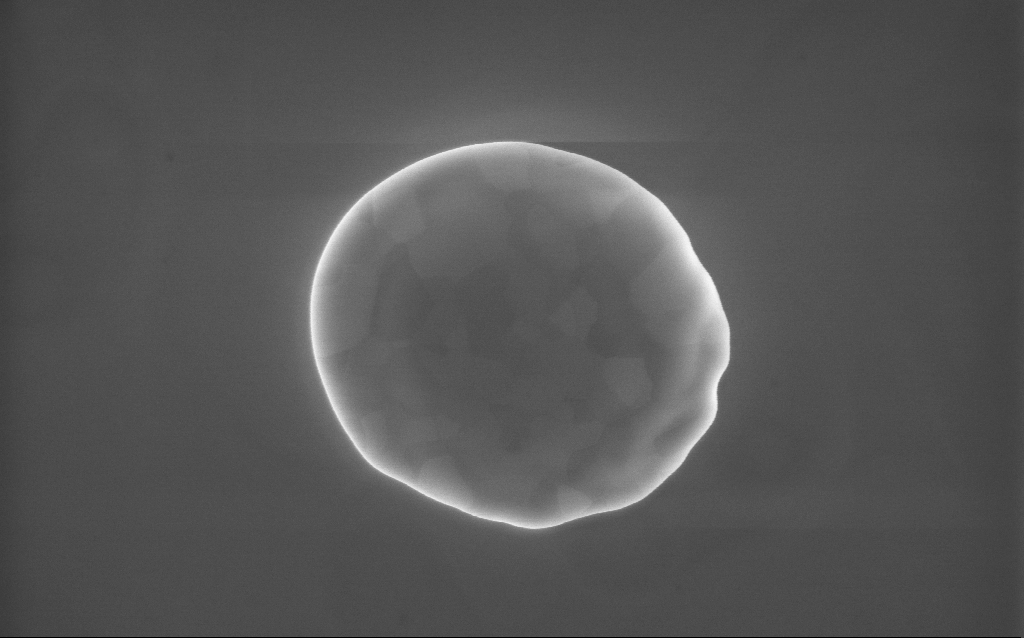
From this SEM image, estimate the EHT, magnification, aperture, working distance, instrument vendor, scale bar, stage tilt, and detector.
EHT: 10 kV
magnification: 63 K X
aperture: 30 µm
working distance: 2 mm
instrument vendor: Zeiss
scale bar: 1000 nm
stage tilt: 0°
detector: InLens